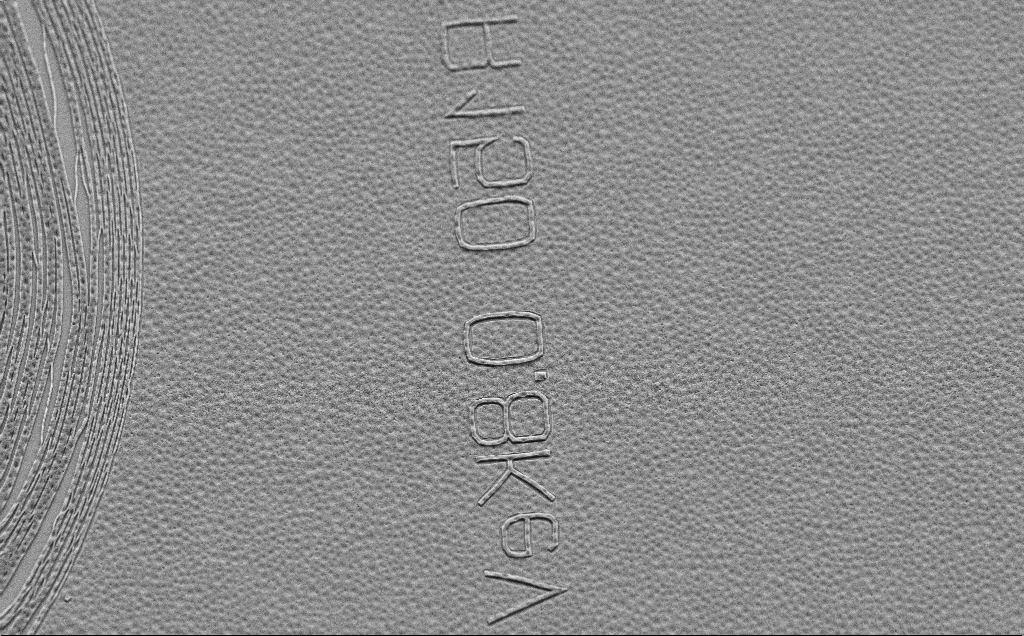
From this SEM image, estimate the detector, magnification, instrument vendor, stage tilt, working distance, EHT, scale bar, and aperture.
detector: SE2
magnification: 3.25 K X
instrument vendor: Zeiss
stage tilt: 45°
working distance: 6 mm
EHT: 5 kV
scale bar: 10000 nm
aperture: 30 µm